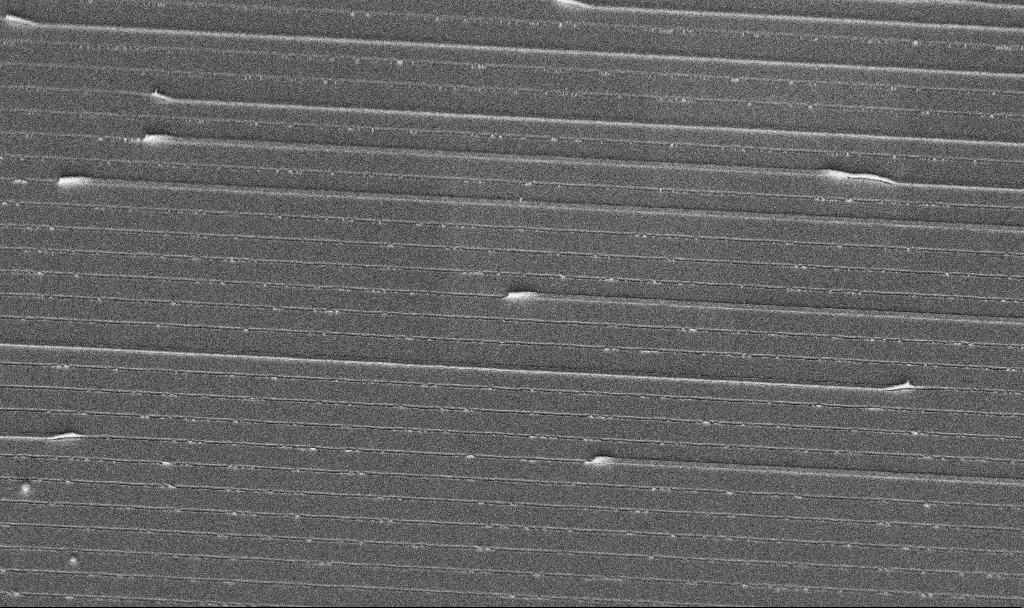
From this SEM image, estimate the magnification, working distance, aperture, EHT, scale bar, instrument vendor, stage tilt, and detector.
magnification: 9.6 K X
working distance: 7.5 mm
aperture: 30 µm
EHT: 5 kV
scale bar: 2000 nm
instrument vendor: Zeiss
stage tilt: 45°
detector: InLens